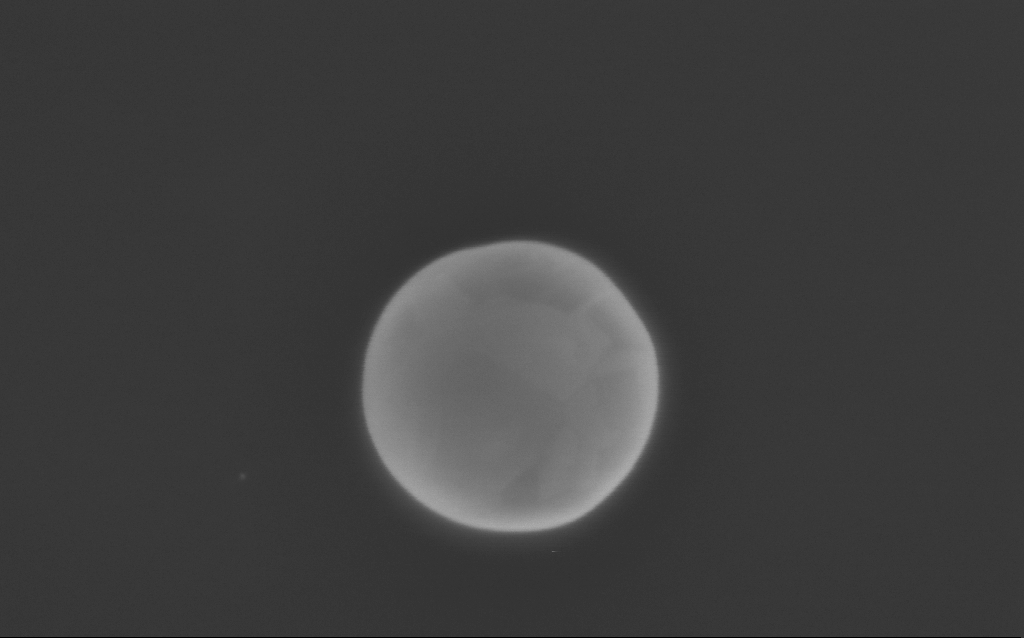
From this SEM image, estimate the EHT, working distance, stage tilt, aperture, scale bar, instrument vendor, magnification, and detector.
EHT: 2 kV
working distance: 3 mm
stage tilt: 0°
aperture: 30 µm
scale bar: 200 nm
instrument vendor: Zeiss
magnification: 168.7 K X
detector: InLens